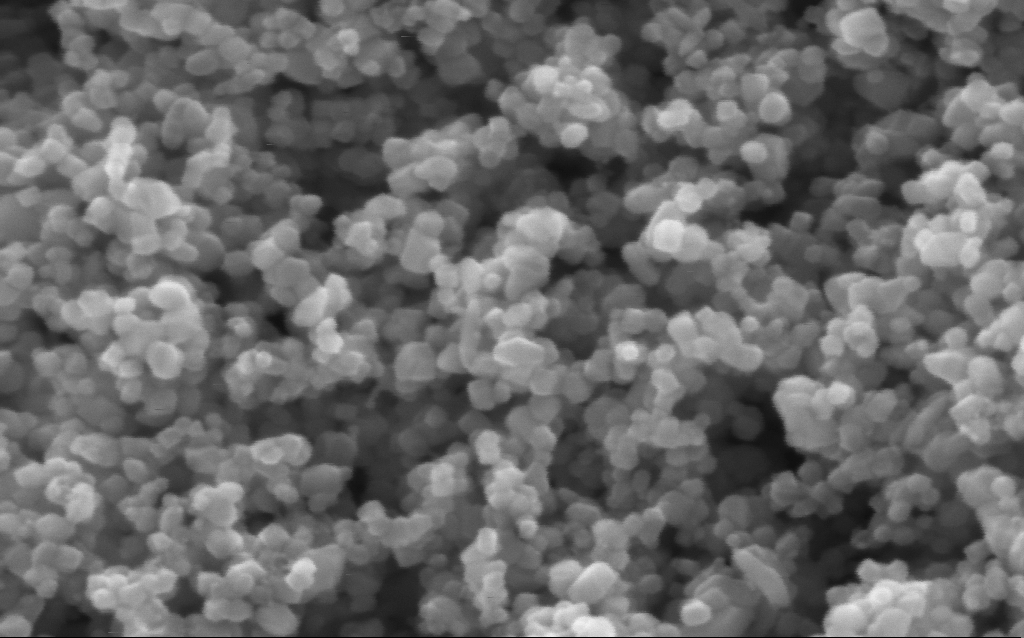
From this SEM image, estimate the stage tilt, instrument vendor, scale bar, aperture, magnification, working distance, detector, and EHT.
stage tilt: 0°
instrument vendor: Zeiss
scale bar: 100 nm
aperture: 30 µm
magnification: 416 K X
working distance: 4.4 mm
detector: InLens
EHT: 5 kV